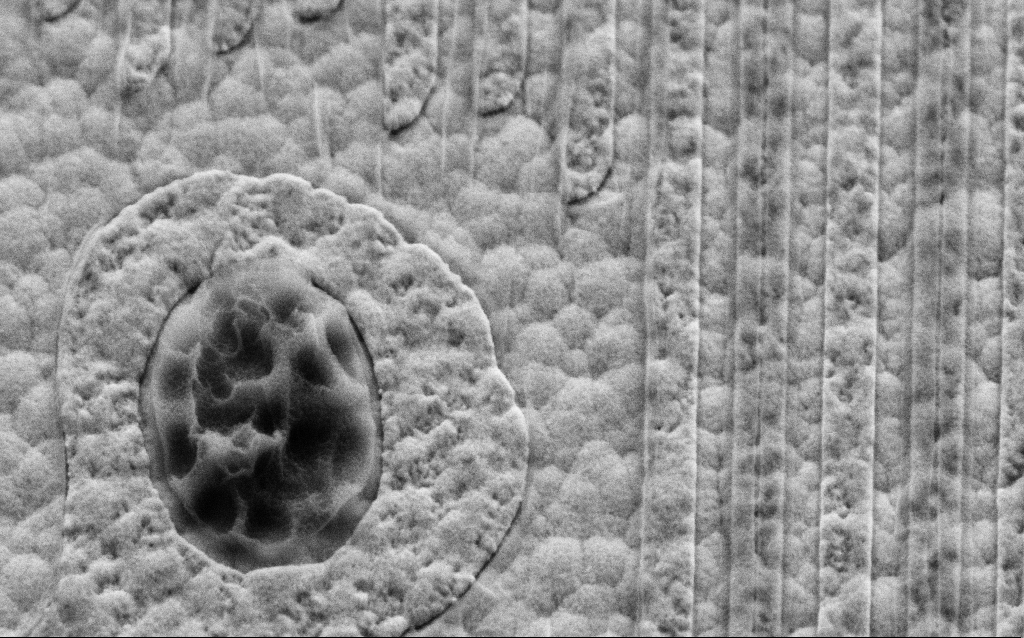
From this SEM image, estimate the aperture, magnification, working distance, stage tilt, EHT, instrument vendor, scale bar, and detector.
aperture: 30 µm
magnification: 65.96 K X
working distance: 6 mm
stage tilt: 45°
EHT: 3 kV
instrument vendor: Zeiss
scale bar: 1000 nm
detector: SE2